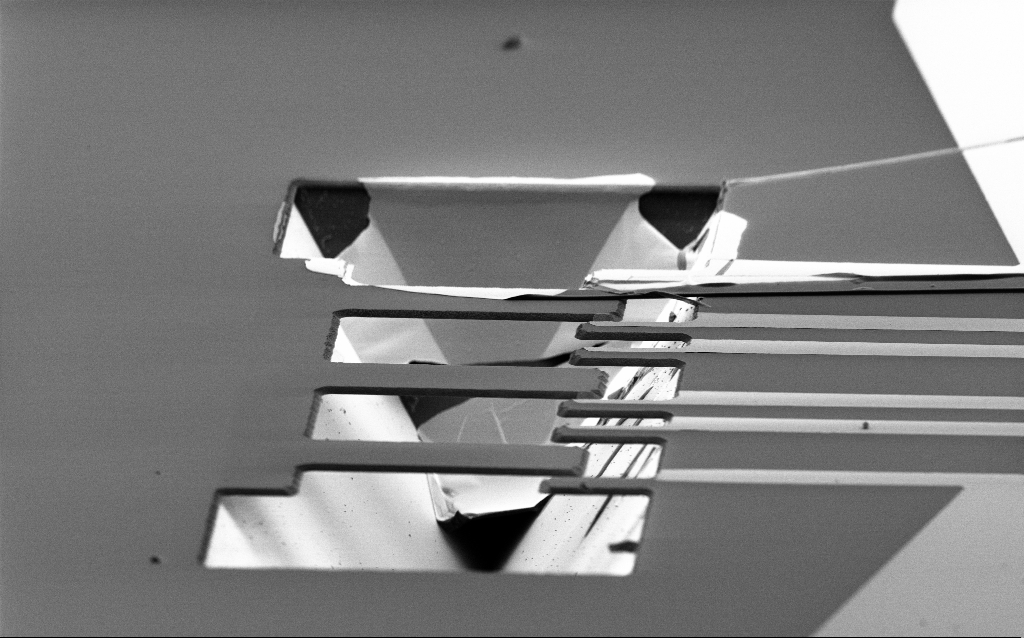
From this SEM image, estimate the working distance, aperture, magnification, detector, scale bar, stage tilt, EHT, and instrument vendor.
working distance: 9 mm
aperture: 30 µm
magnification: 1.38 K X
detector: SE2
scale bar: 20000 nm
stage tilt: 70°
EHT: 2 kV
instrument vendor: Zeiss